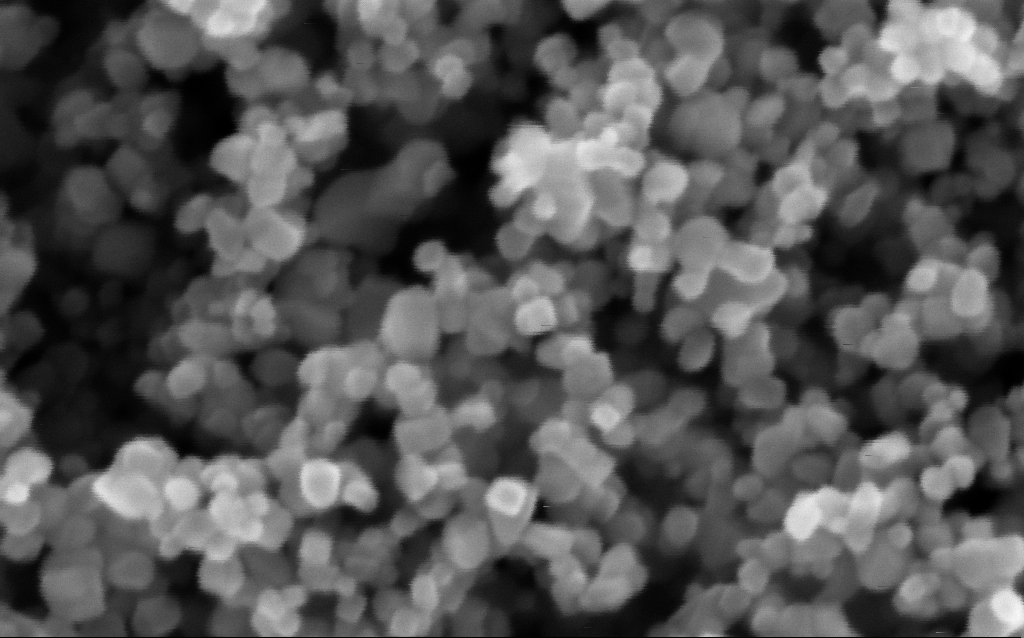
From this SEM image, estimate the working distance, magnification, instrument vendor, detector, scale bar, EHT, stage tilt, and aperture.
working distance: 5.3 mm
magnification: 608.38 K X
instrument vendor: Zeiss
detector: InLens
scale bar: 100 nm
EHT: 5 kV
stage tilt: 0°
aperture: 30 µm